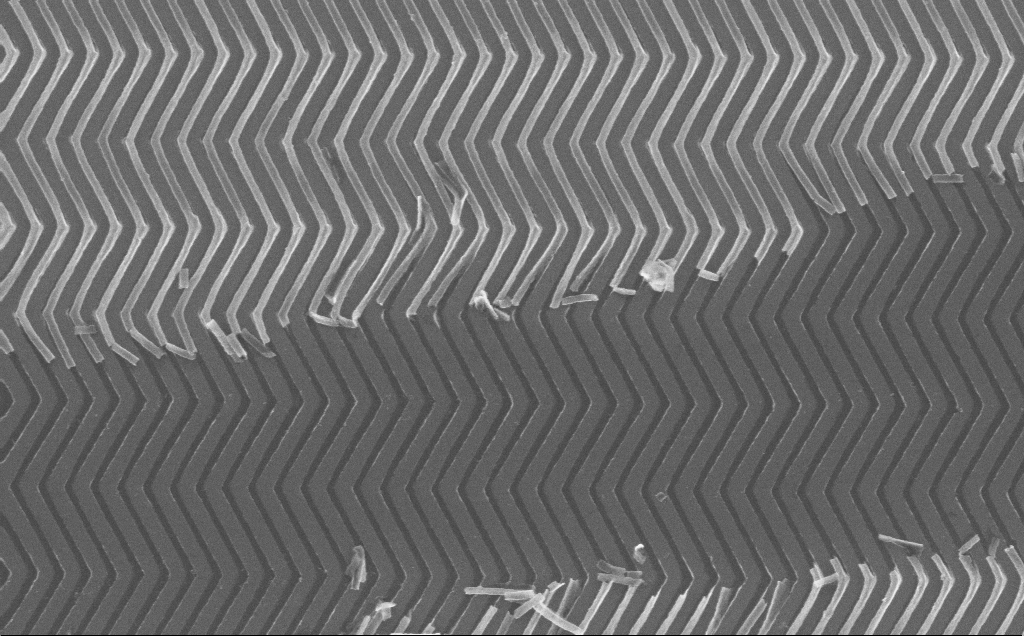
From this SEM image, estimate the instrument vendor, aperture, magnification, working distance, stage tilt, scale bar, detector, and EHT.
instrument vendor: Zeiss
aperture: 30 µm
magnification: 32.13 K X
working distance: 7 mm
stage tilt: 0°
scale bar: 2000 nm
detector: InLens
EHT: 10 kV